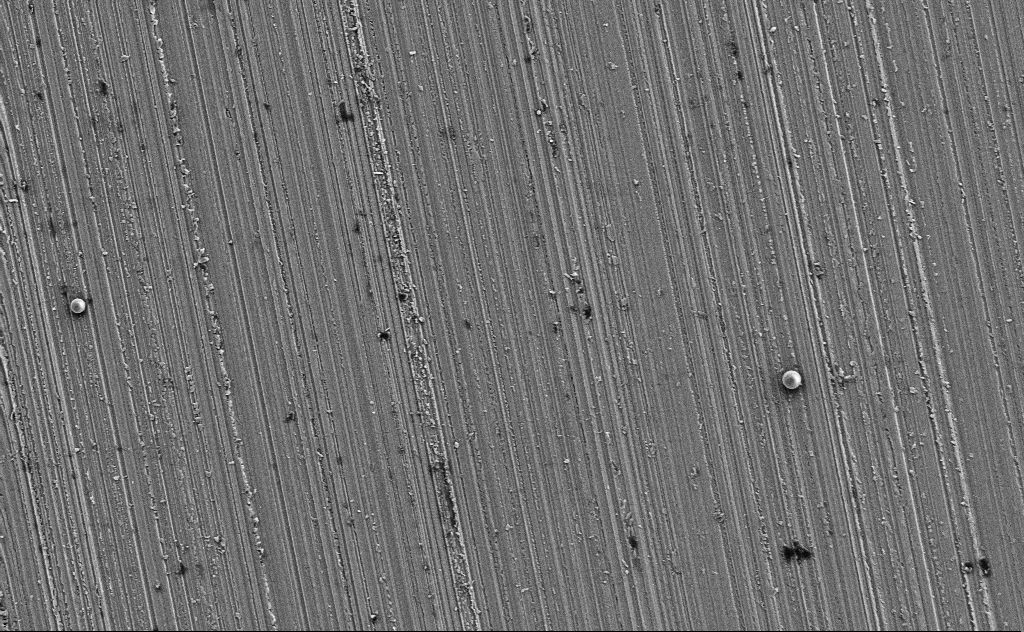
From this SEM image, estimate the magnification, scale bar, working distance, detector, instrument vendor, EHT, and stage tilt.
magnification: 5.4 K X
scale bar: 10000 nm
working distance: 14 mm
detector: SE2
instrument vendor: Zeiss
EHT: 3 kV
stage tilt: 0°